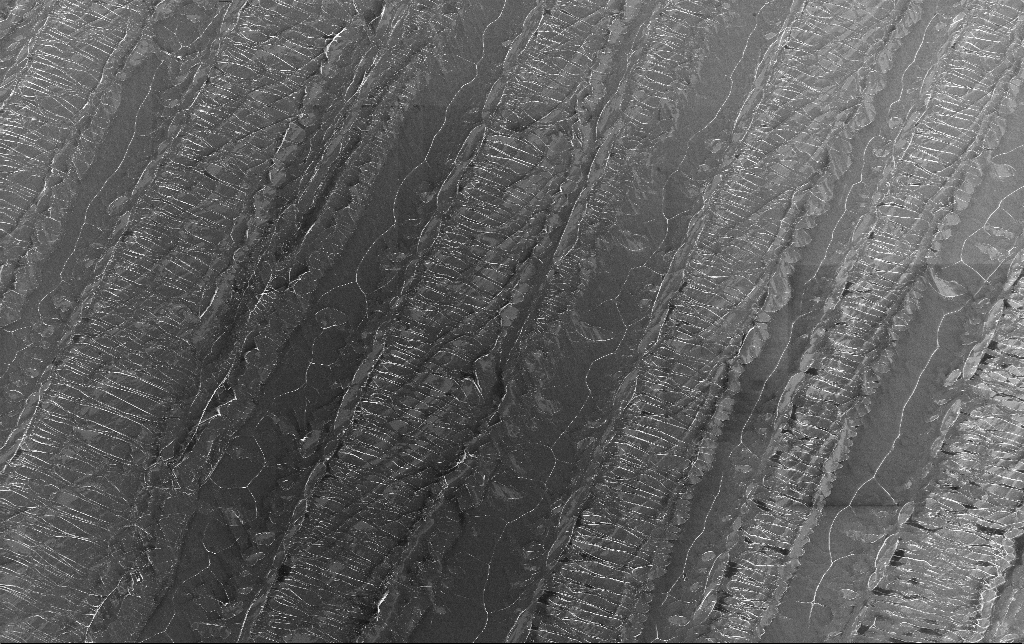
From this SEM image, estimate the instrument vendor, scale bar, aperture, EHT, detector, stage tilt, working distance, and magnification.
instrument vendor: Zeiss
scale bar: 100000 nm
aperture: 30 µm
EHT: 5 kV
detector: InLens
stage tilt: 0°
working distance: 3.8 mm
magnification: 0.545 K X